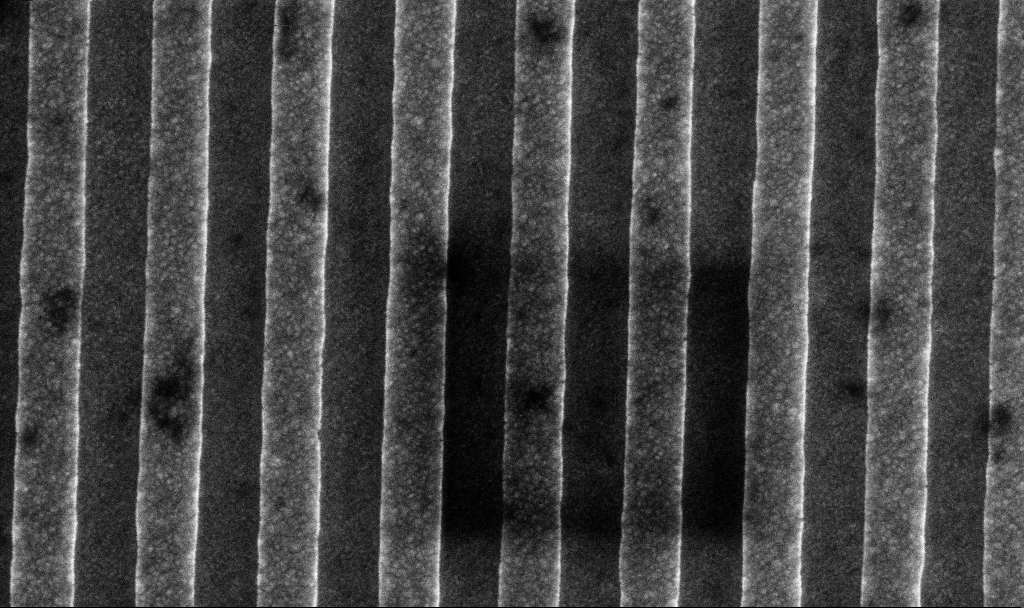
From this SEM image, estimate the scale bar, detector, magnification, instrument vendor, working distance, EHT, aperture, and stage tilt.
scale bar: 200 nm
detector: InLens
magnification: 109.05 K X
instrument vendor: Zeiss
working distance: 5.6 mm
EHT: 10 kV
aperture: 60 µm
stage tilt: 0°